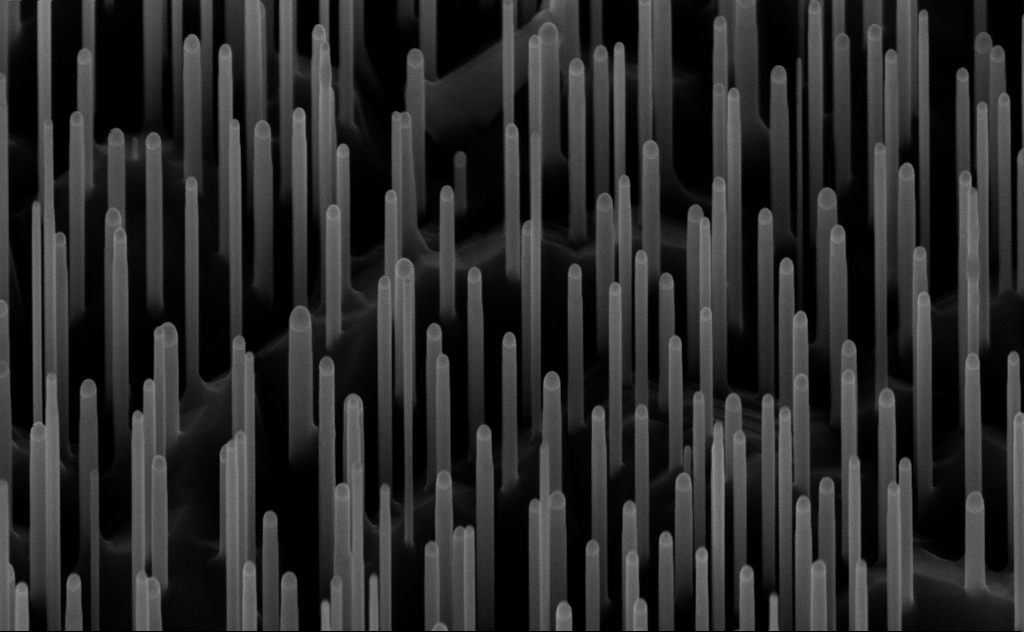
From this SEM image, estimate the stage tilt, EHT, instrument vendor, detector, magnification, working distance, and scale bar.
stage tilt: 45°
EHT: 10 kV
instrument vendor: Zeiss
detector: InLens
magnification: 80 K X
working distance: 7 mm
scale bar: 200 nm